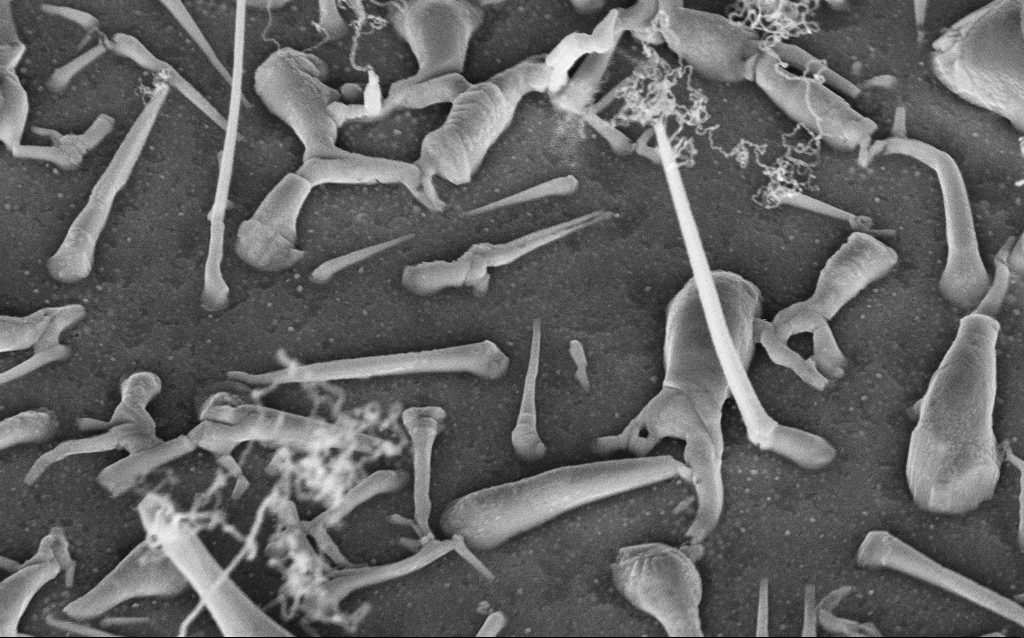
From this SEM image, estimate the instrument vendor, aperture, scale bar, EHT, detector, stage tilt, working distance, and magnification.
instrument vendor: Zeiss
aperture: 30 µm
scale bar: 1000 nm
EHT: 5 kV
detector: InLens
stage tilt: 42°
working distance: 8 mm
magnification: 60.63 K X